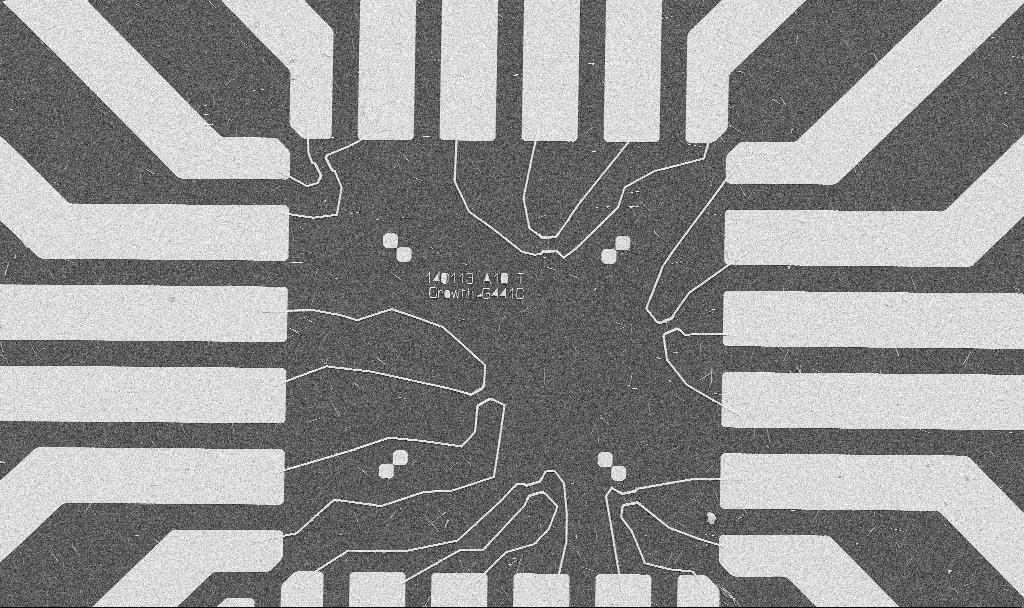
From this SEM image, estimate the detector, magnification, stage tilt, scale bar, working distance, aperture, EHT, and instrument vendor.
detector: SE2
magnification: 1 K X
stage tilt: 0°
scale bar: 20000 nm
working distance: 10.7 mm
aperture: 30 µm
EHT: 5 kV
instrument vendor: Zeiss